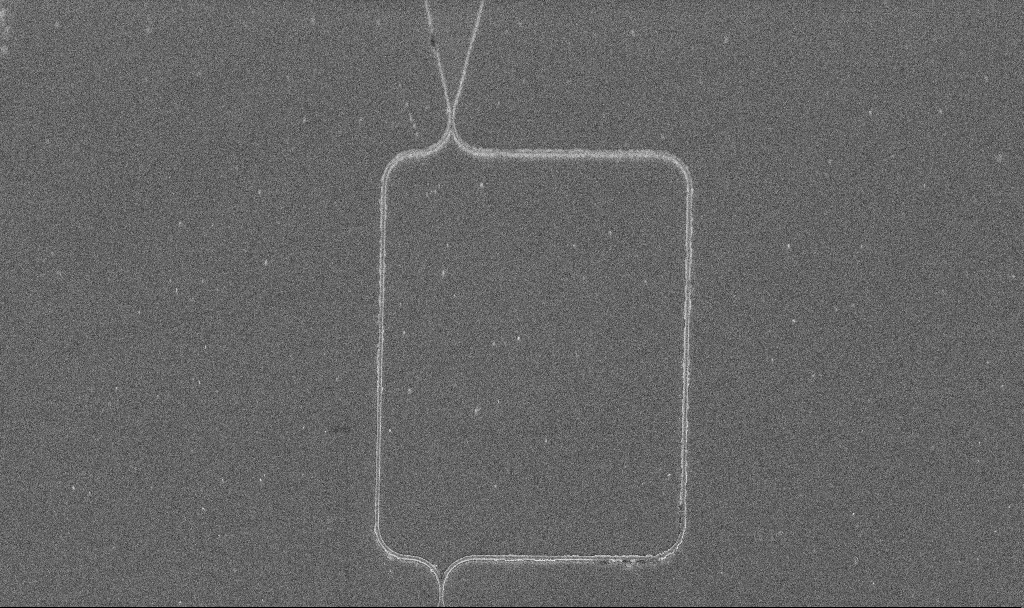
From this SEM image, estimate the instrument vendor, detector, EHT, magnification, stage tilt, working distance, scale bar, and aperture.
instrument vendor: Zeiss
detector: InLens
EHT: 5 kV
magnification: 2.57 K X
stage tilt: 45°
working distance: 9.5 mm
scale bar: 20000 nm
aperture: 30 µm